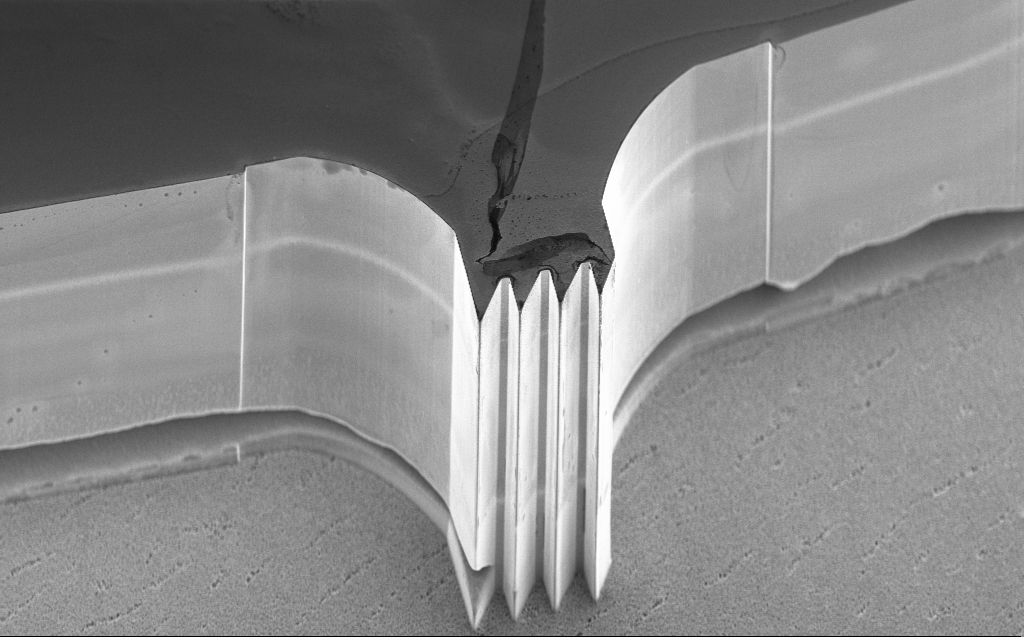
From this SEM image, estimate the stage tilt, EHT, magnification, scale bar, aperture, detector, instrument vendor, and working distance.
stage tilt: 45°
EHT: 5 kV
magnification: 1.36 K X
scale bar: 20000 nm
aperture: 30 µm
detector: InLens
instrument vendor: Zeiss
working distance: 5 mm